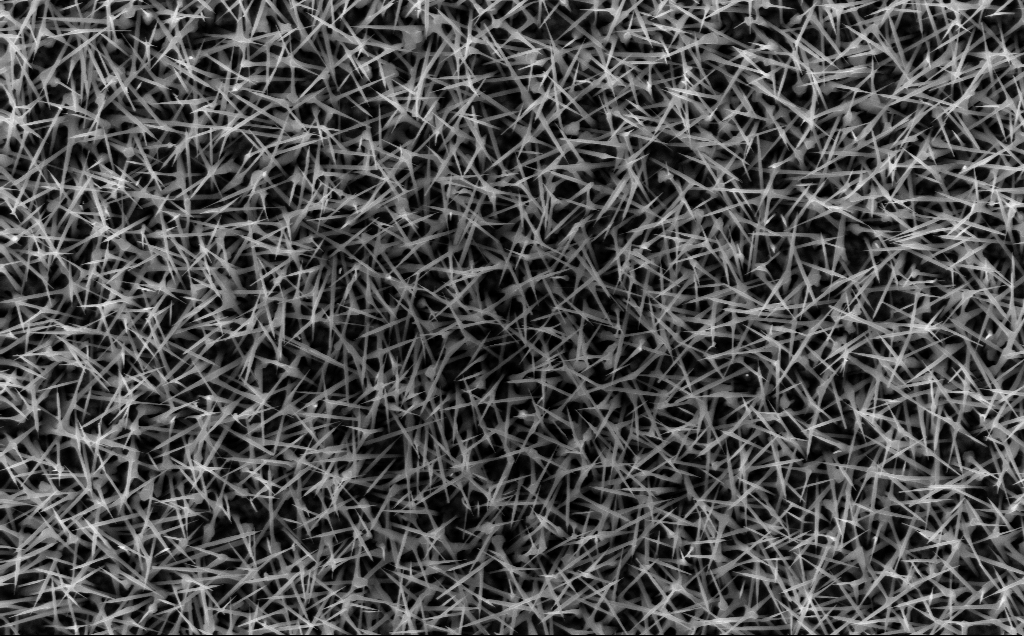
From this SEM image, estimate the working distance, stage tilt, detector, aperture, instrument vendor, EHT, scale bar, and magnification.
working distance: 7 mm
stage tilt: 0°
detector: InLens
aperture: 30 µm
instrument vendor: Zeiss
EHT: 10 kV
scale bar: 1000 nm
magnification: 20 K X